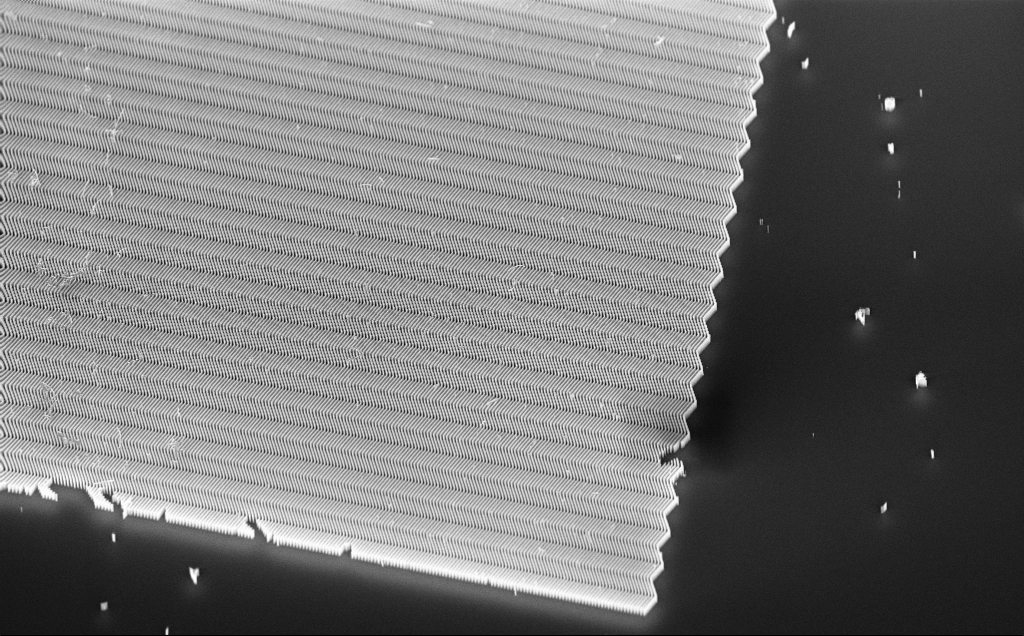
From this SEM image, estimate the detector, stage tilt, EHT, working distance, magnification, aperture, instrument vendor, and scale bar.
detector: InLens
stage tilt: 45°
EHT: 10 kV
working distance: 6 mm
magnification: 6.12 K X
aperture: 30 µm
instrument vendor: Zeiss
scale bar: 10000 nm